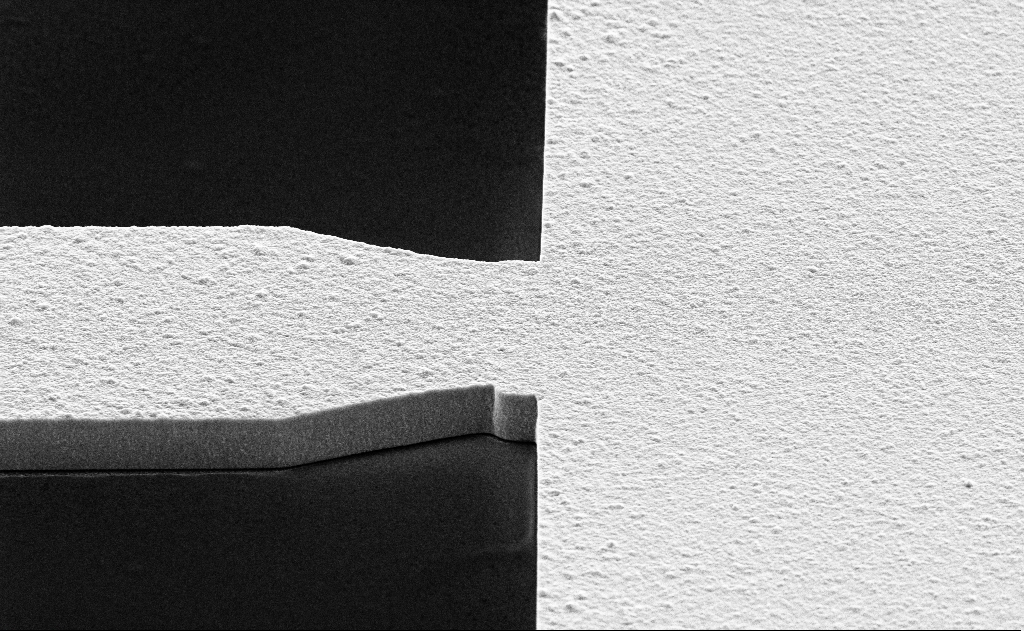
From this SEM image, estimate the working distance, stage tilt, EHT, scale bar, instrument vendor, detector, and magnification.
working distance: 13 mm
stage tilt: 60°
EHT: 10 kV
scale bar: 1000 nm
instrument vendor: Zeiss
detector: SE2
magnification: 12.08 K X